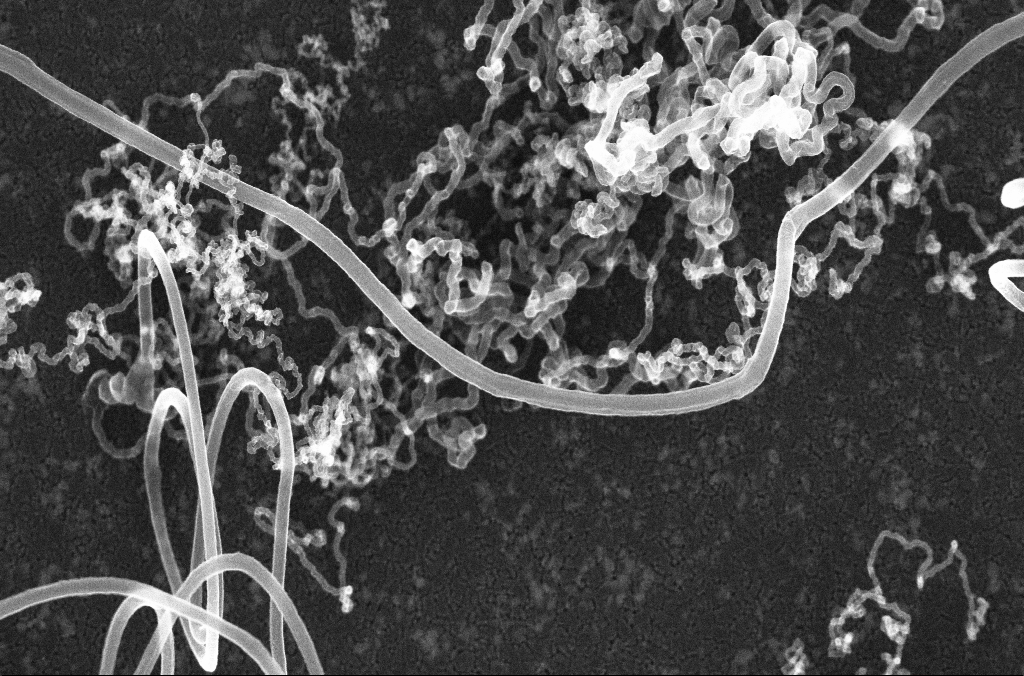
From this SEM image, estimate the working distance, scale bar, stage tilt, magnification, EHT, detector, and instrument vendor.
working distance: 4.4 mm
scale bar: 1000 nm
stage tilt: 0°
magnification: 50 K X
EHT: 20 kV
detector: InLens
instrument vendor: Zeiss